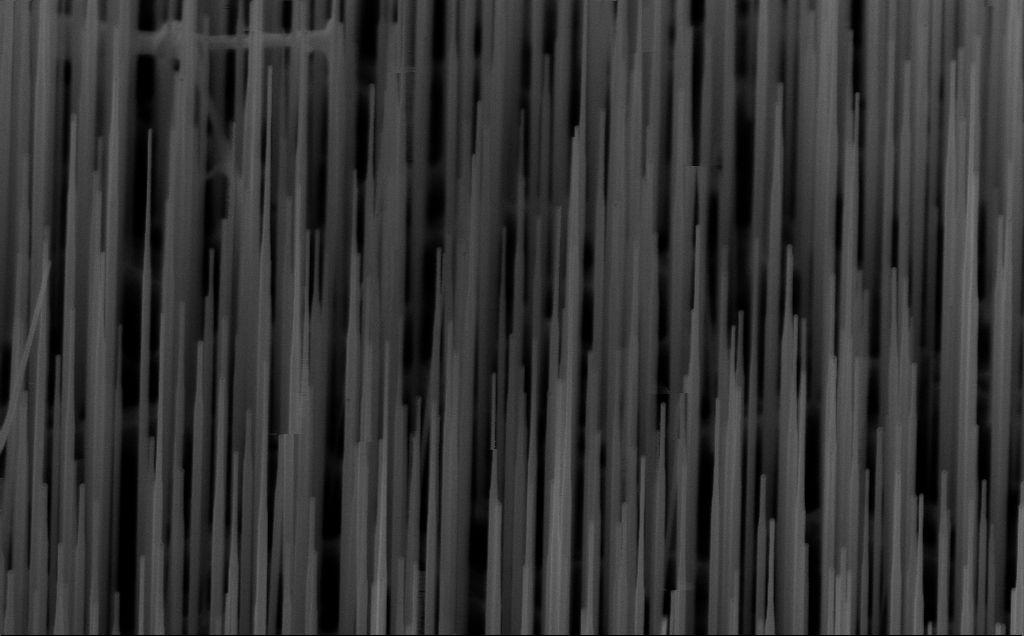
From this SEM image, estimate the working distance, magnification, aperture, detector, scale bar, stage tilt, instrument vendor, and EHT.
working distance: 6 mm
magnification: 40 K X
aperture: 30 µm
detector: InLens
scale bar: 1000 nm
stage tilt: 45°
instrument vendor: Zeiss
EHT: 10 kV